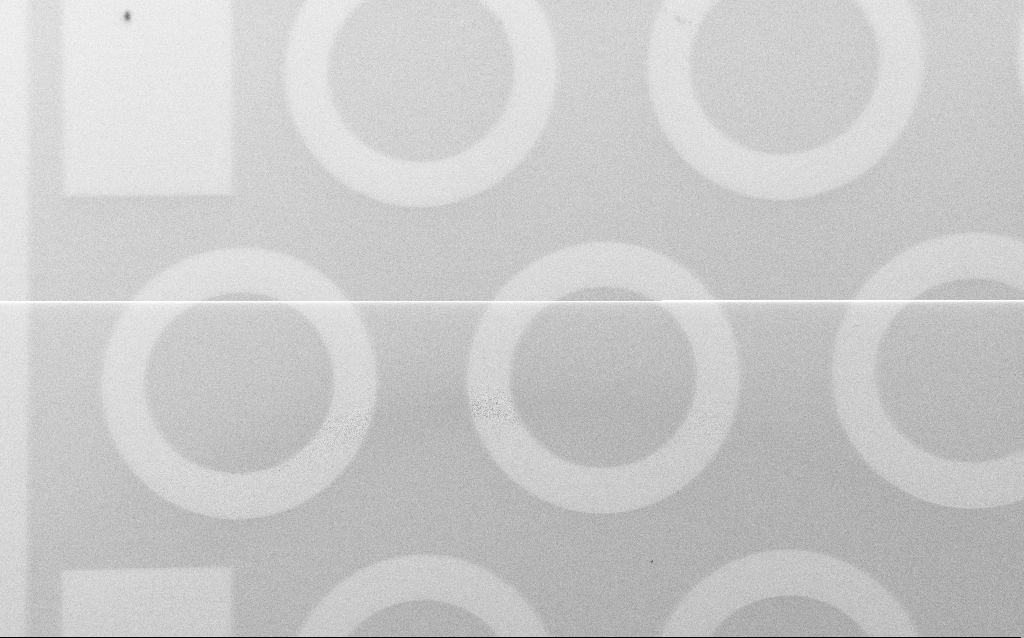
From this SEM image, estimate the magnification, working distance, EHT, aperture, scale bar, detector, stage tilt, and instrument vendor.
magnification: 0.211 K X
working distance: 8 mm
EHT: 3 kV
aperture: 30 µm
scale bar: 100000 nm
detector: SE2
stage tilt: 45°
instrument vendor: Zeiss